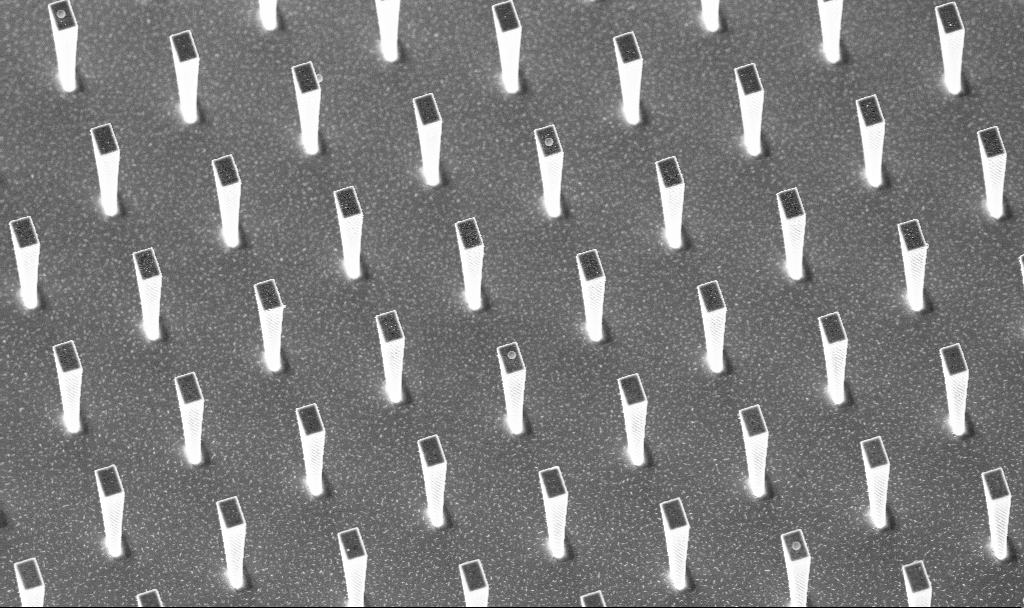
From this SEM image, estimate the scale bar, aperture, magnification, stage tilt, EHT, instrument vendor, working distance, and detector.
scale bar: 10000 nm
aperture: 30 µm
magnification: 4.18 K X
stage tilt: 20°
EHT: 5 kV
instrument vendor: Zeiss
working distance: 5.2 mm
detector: InLens